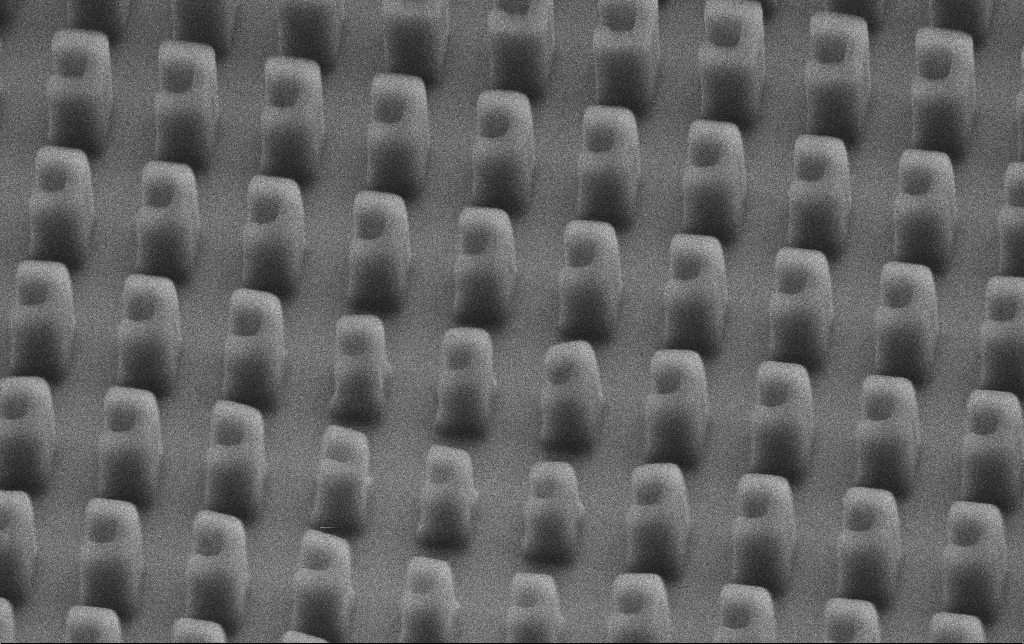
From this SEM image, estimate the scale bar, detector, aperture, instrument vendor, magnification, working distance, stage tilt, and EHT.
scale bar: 1000 nm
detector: SE2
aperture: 30 µm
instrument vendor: Zeiss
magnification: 39.51 K X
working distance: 7 mm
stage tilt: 45°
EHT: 3 kV